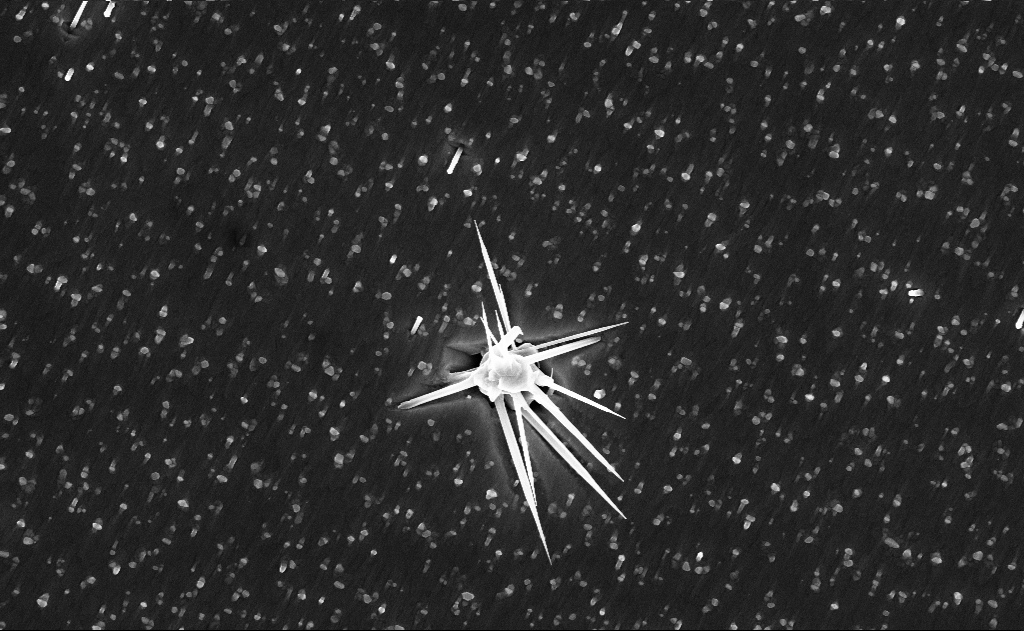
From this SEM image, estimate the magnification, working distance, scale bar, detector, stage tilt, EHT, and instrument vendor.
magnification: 20 K X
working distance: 9 mm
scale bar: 1000 nm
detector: InLens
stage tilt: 0°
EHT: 10 kV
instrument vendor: Zeiss